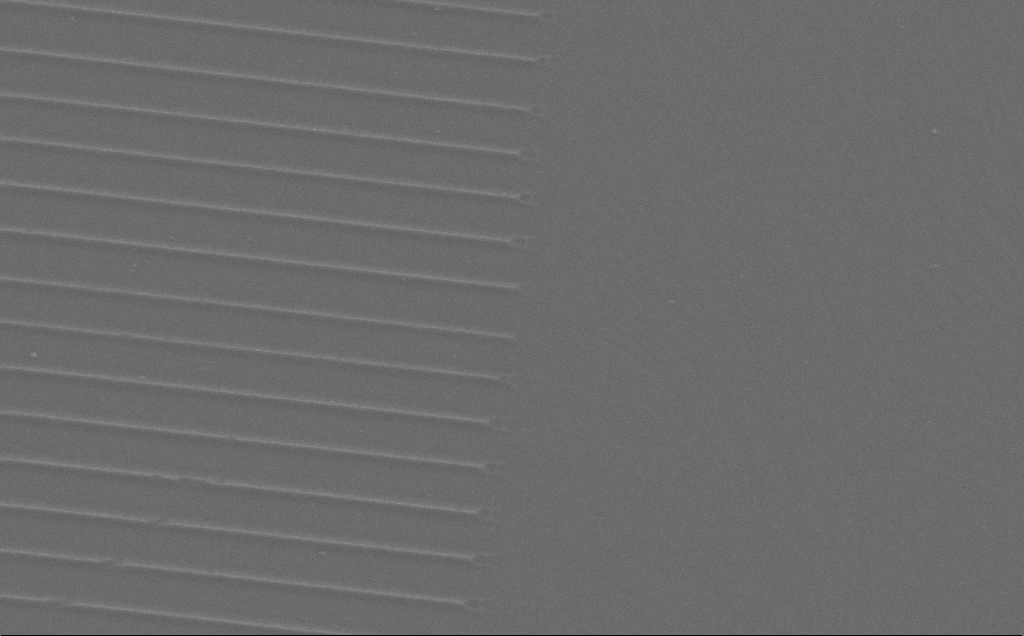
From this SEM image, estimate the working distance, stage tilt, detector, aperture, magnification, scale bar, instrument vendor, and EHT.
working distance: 11 mm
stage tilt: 0°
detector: SE2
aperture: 30 µm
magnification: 2.56 K X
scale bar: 10000 nm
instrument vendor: Zeiss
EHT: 10 kV